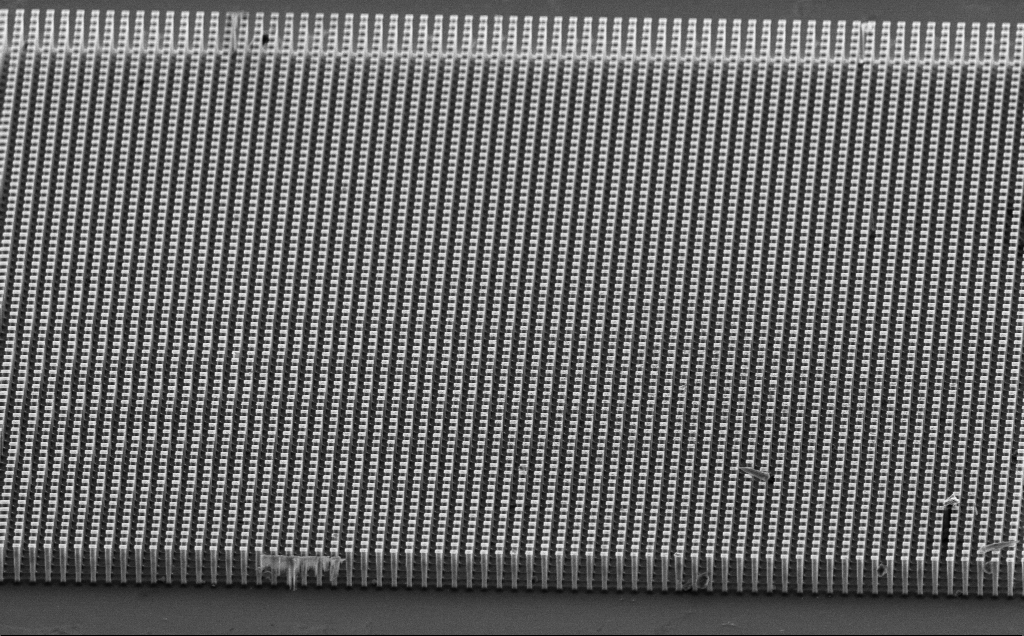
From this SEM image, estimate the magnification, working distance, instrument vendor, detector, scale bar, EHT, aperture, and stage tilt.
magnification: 1.38 K X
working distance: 15 mm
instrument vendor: Zeiss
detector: SE2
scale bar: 10000 nm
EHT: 10 kV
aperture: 30 µm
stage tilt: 60.5°